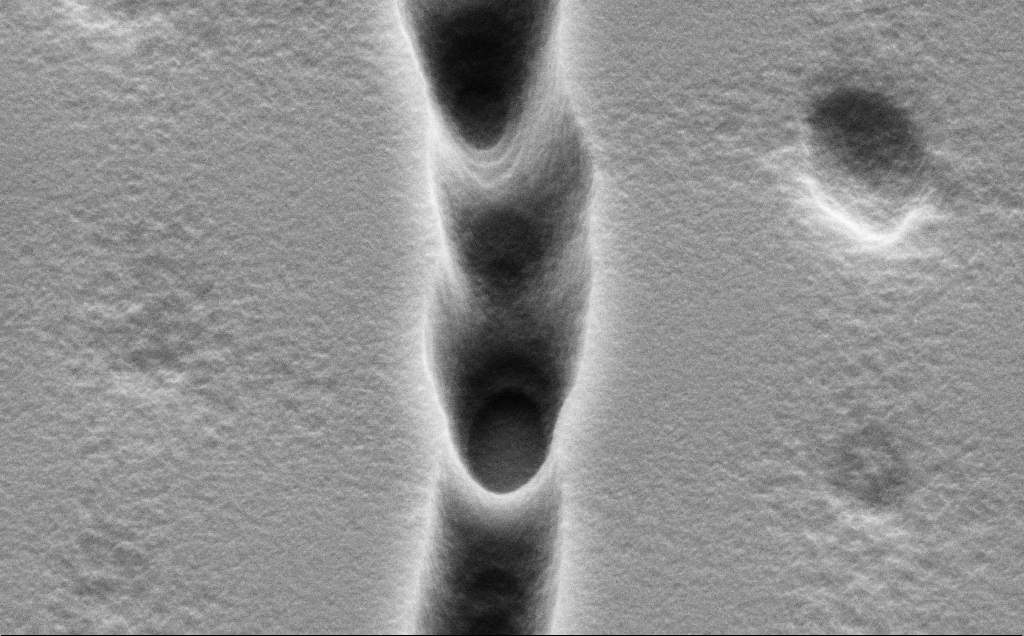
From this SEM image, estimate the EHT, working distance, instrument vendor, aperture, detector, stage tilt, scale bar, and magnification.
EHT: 5 kV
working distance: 10 mm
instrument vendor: Zeiss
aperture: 30 µm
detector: SE2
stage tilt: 45°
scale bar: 1000 nm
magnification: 42.04 K X